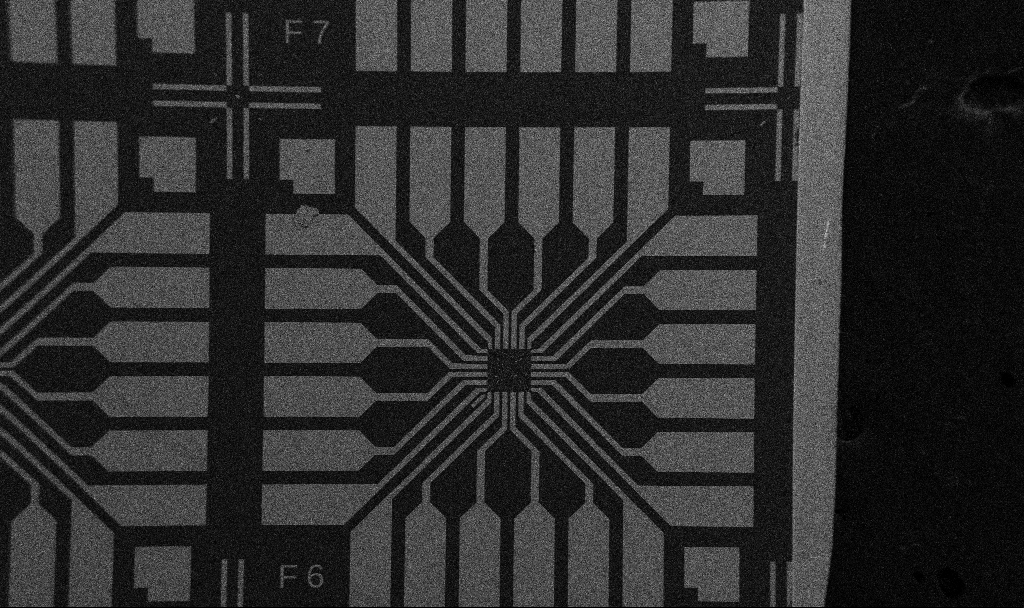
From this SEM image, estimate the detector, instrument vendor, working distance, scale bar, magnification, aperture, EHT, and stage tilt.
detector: SE2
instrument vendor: Zeiss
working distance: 10.7 mm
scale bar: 200000 nm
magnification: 0.1 K X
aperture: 30 µm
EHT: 5 kV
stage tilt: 0°